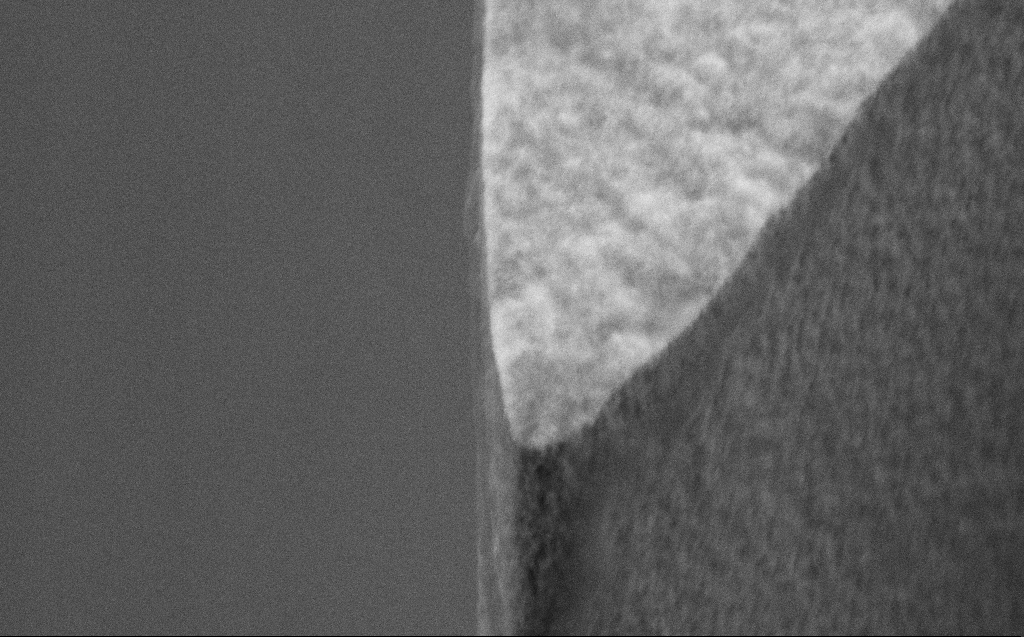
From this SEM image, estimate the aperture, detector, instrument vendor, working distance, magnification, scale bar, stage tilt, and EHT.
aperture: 30 µm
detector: SE2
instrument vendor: Zeiss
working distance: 5 mm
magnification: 66.21 K X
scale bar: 1000 nm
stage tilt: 45°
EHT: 5 kV